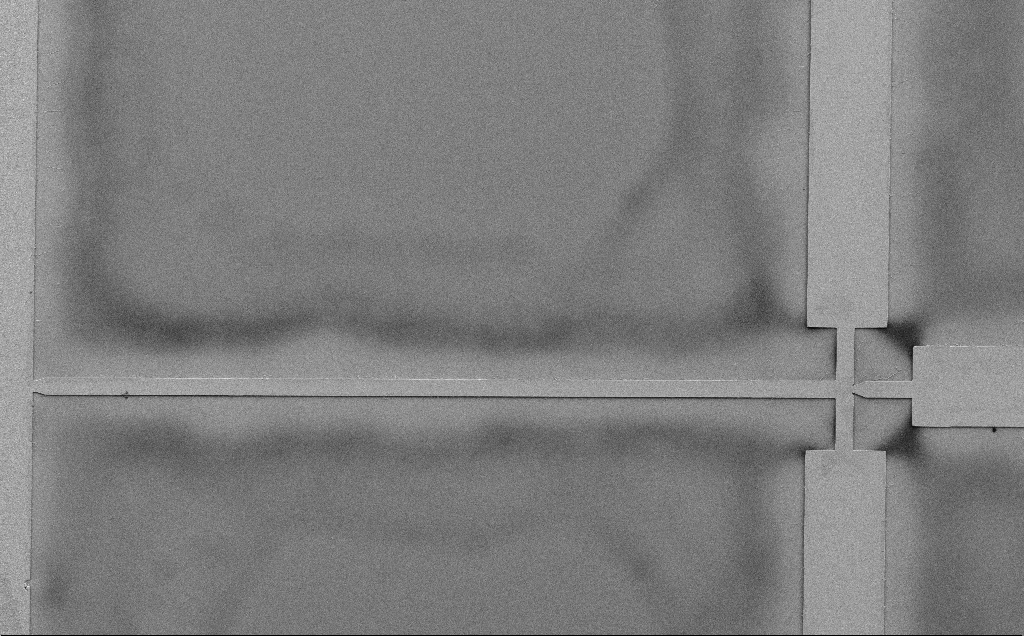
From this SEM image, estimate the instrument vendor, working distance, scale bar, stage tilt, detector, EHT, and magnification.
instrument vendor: Zeiss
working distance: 7 mm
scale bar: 100000 nm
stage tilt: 0°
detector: SE2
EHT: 5 kV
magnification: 0.582 K X